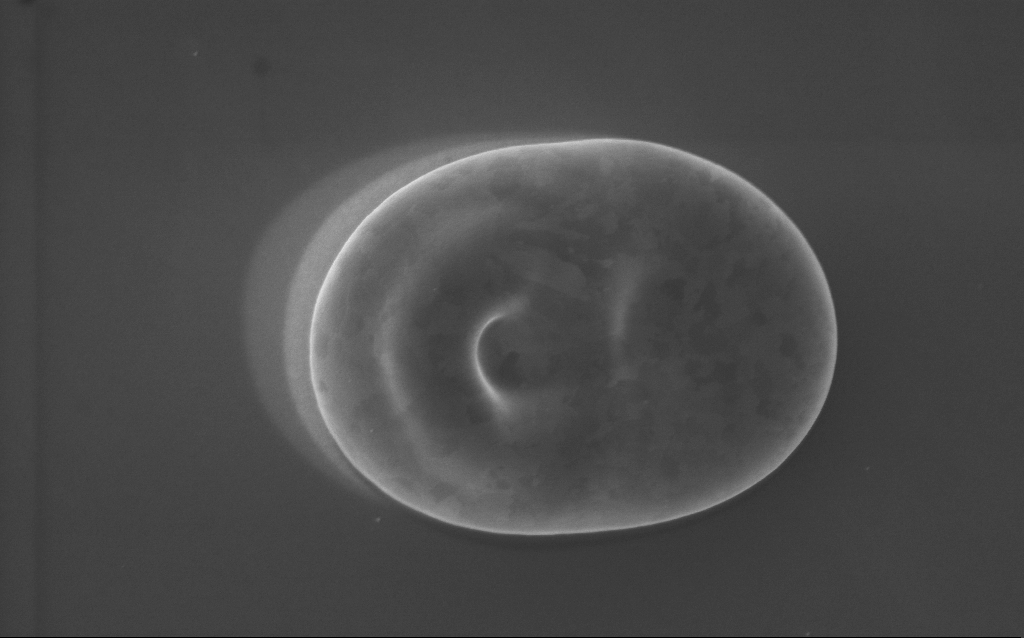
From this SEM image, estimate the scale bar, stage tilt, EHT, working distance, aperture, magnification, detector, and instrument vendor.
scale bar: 1000 nm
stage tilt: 0°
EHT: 5 kV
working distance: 3 mm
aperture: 30 µm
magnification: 38 K X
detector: InLens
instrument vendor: Zeiss